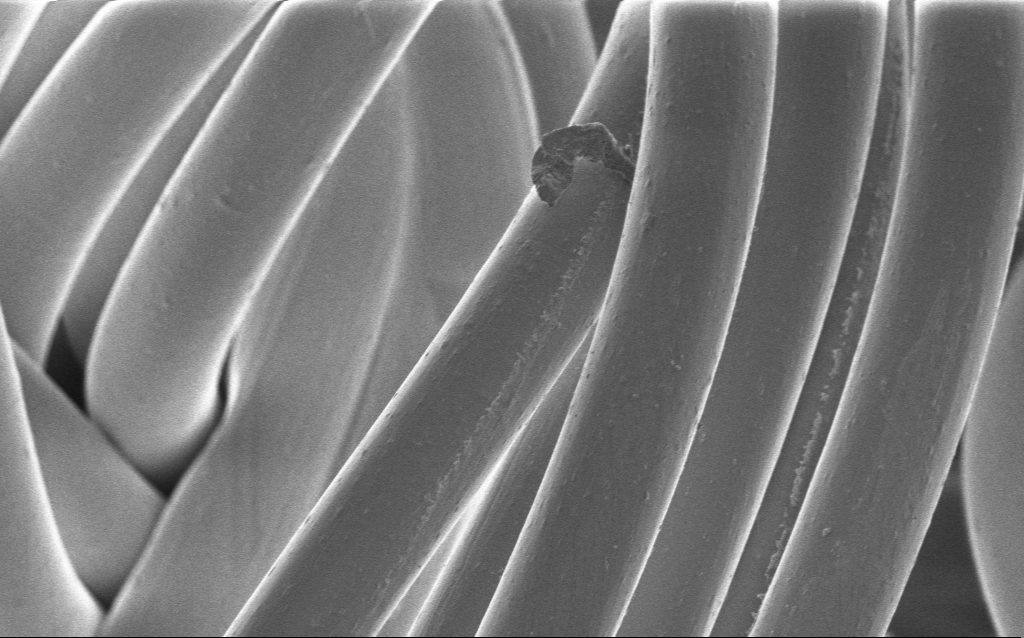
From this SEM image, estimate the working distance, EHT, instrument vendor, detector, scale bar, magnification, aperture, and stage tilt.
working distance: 4 mm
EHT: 1 kV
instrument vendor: Zeiss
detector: InLens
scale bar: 20000 nm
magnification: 1.94 K X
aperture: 30 µm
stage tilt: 0°